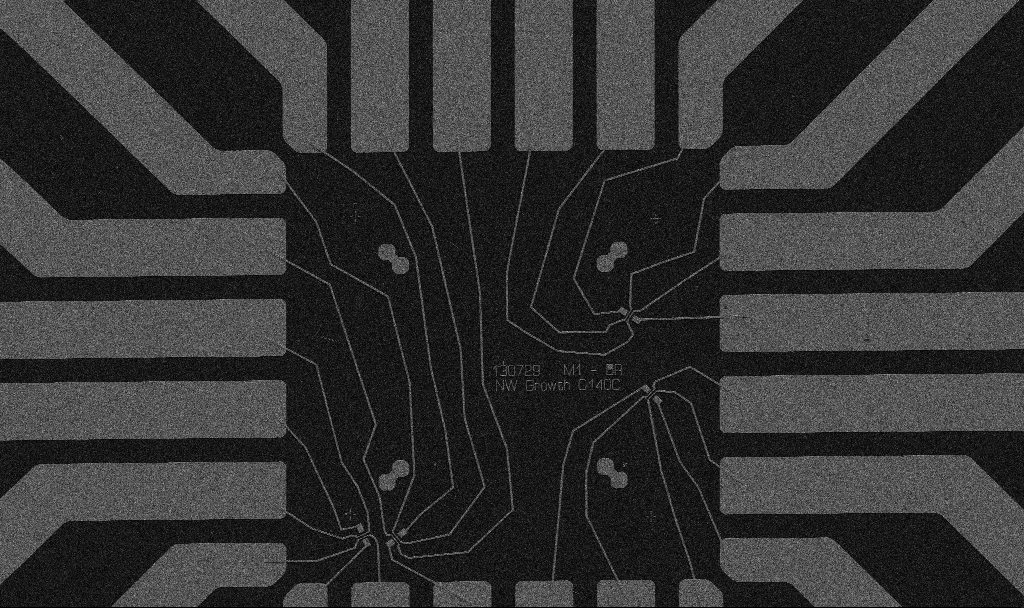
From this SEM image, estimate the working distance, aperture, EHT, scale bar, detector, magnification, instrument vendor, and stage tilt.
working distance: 10.7 mm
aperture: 30 µm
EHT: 5 kV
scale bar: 20000 nm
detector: SE2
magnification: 1 K X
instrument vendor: Zeiss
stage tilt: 0°